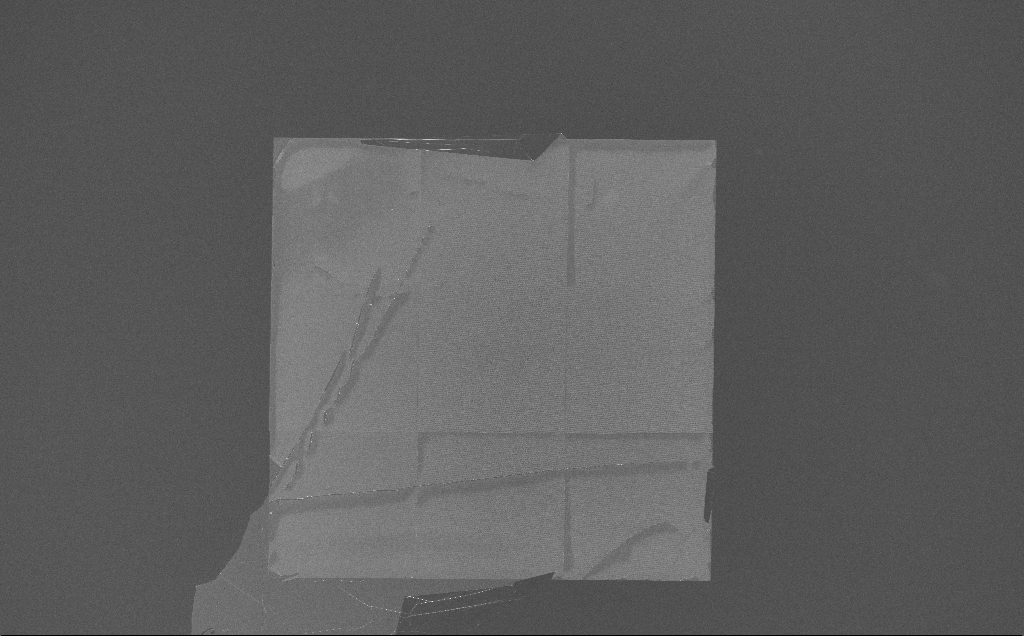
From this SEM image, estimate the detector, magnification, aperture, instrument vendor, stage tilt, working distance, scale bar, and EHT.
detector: InLens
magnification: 0.22 K X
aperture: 30 µm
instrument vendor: Zeiss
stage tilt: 0°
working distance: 7 mm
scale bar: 100000 nm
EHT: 10 kV